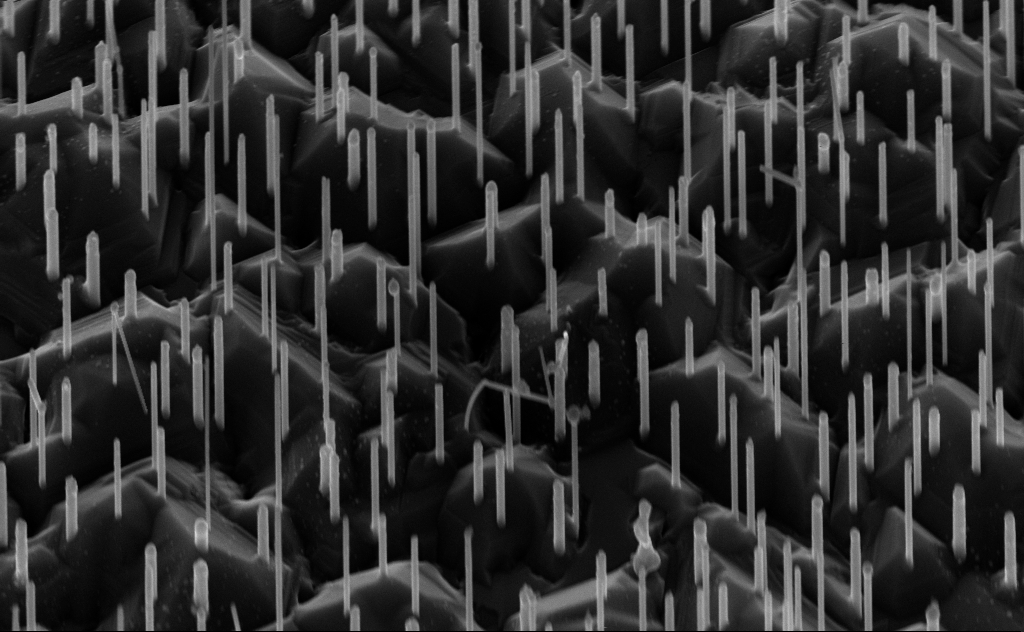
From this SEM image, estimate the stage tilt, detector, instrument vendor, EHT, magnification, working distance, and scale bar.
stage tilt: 45°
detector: InLens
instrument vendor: Zeiss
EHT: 10 kV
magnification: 40 K X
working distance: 6 mm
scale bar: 1000 nm